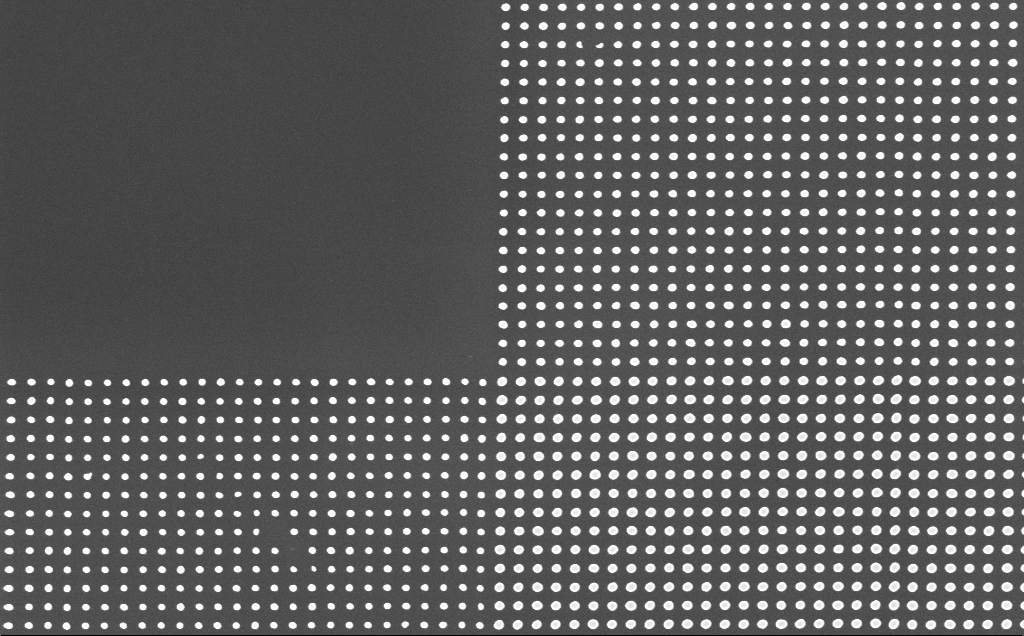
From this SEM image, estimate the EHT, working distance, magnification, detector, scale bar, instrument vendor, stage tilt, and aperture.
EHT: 10 kV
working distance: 6 mm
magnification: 34.62 K X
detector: InLens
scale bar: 1000 nm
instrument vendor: Zeiss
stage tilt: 0°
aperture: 30 µm